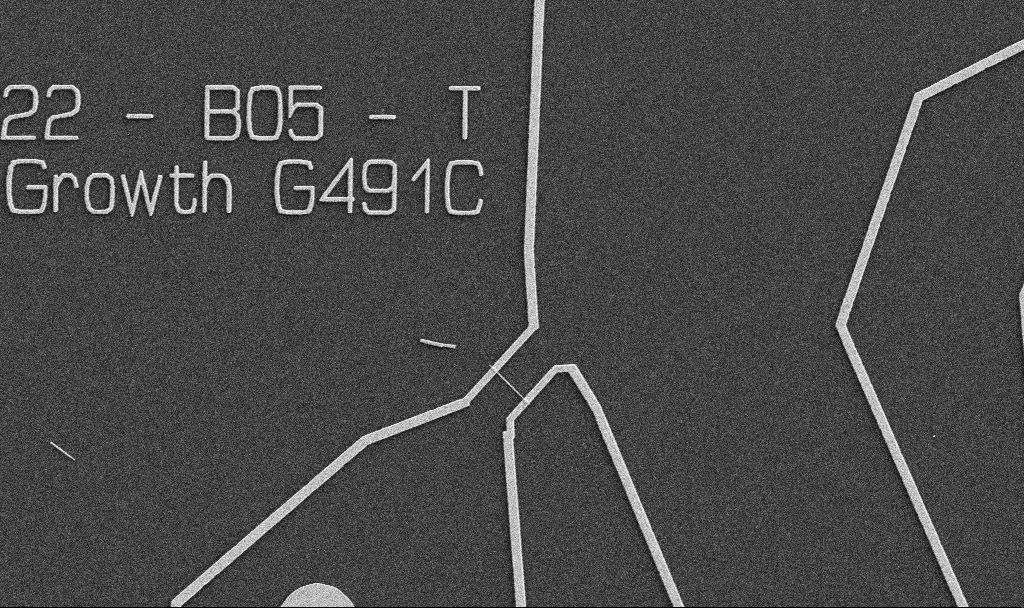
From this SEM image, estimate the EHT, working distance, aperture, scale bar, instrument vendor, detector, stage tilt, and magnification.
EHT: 5 kV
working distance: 10.7 mm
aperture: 30 µm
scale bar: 10000 nm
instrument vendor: Zeiss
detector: SE2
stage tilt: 0°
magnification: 5 K X